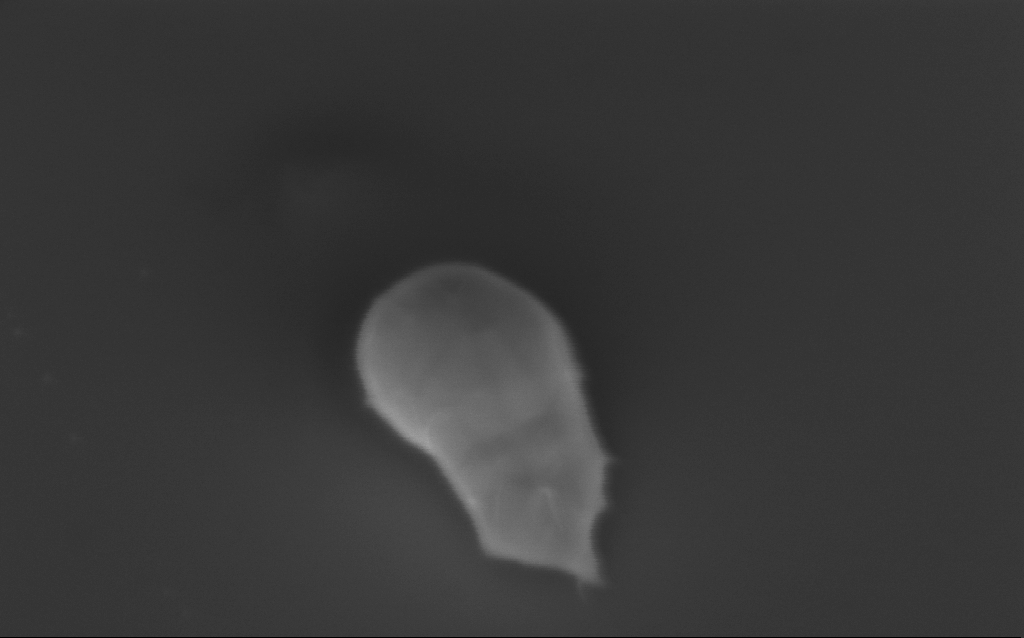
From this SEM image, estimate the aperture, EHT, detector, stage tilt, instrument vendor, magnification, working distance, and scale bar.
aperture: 30 µm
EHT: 3 kV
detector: InLens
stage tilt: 40°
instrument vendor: Zeiss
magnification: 211.12 K X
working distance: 5 mm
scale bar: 200 nm